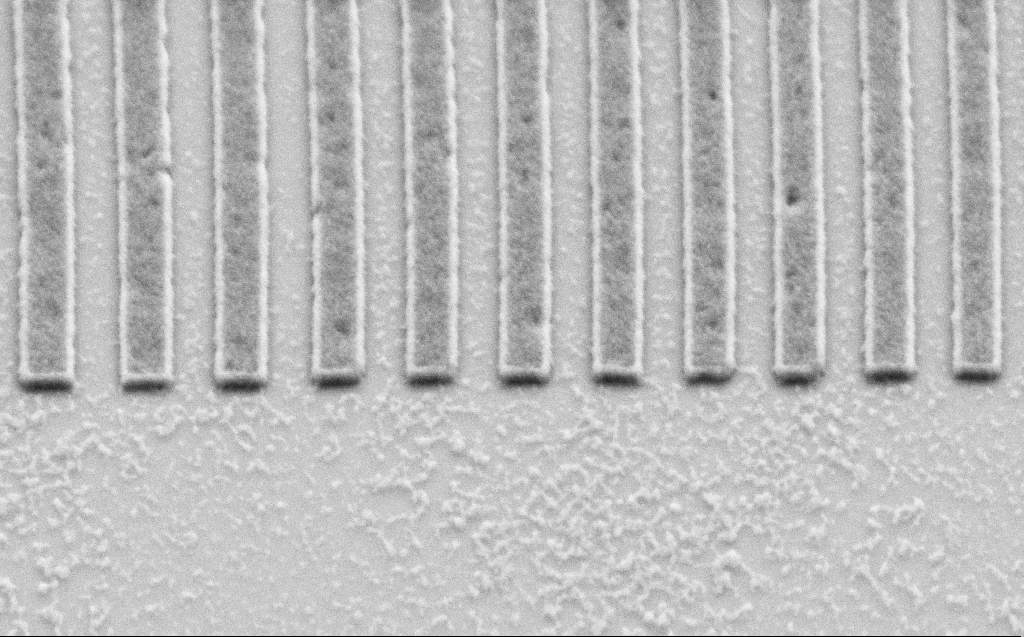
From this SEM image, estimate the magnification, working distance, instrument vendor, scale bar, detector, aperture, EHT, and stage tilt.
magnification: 45.48 K X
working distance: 6 mm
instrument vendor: Zeiss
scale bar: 1000 nm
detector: SE2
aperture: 30 µm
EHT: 2.5 kV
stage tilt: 44.1°